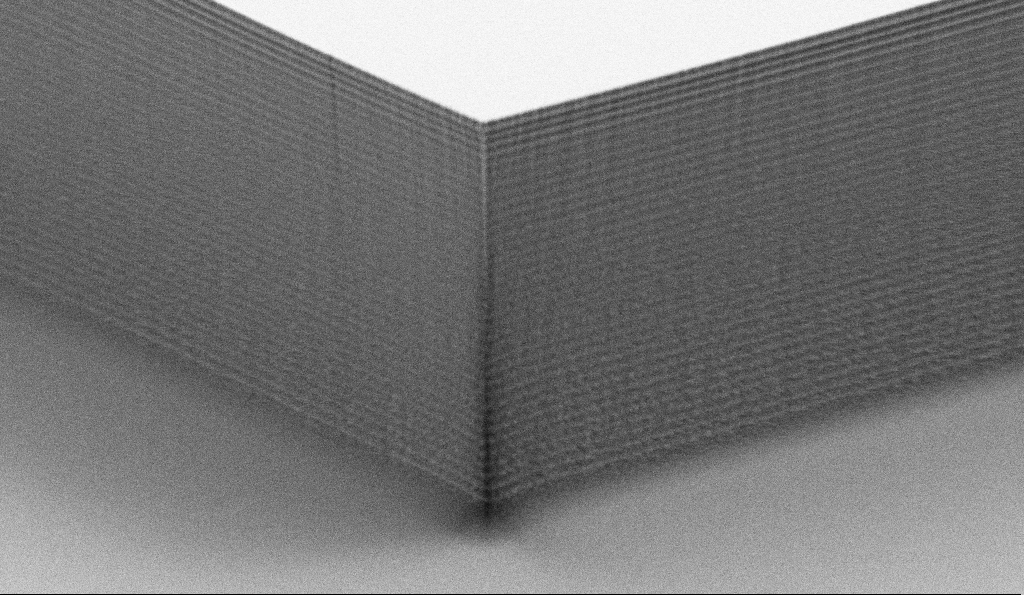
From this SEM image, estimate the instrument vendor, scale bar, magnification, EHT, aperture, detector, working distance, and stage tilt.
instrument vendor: Zeiss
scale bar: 2000 nm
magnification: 9.15 K X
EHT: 5 kV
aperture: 30 µm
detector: SE2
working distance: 4.4 mm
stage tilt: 70°